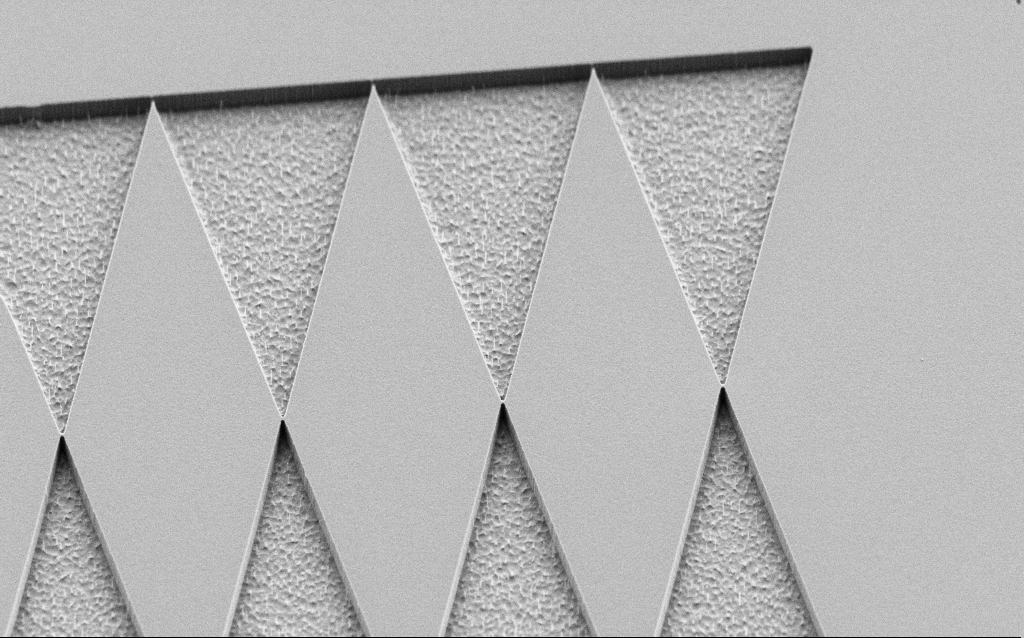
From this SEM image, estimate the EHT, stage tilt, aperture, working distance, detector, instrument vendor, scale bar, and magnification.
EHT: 2 kV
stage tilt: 45°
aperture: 30 µm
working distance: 6 mm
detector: SE2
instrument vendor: Zeiss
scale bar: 2000 nm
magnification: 8.15 K X